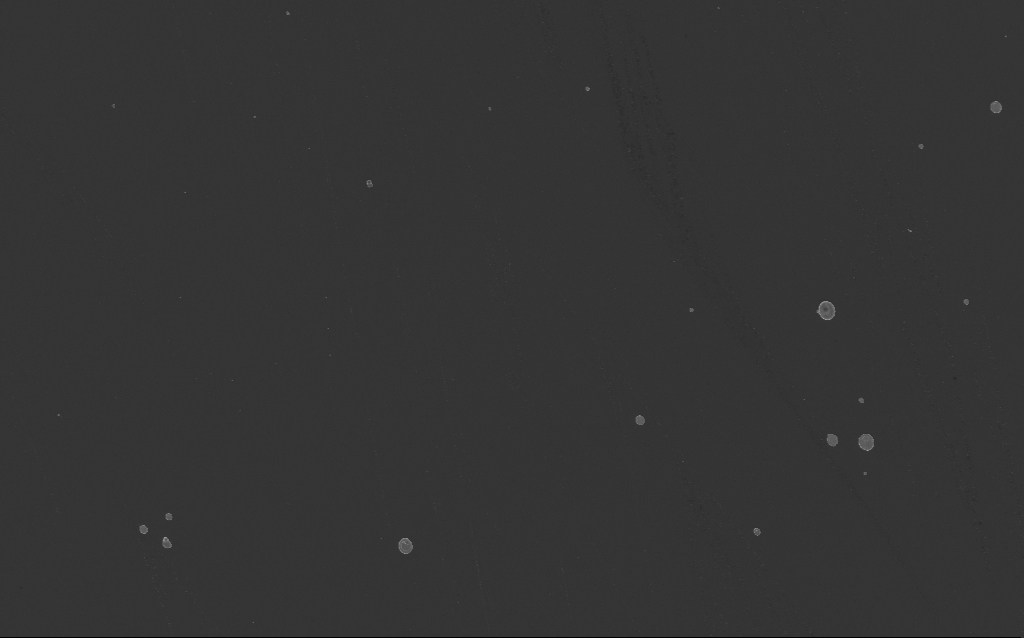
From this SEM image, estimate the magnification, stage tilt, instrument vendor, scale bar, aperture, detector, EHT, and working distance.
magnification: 3.58 K X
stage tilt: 0°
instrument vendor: Zeiss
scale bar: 10000 nm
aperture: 30 µm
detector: InLens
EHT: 3 kV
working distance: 4 mm